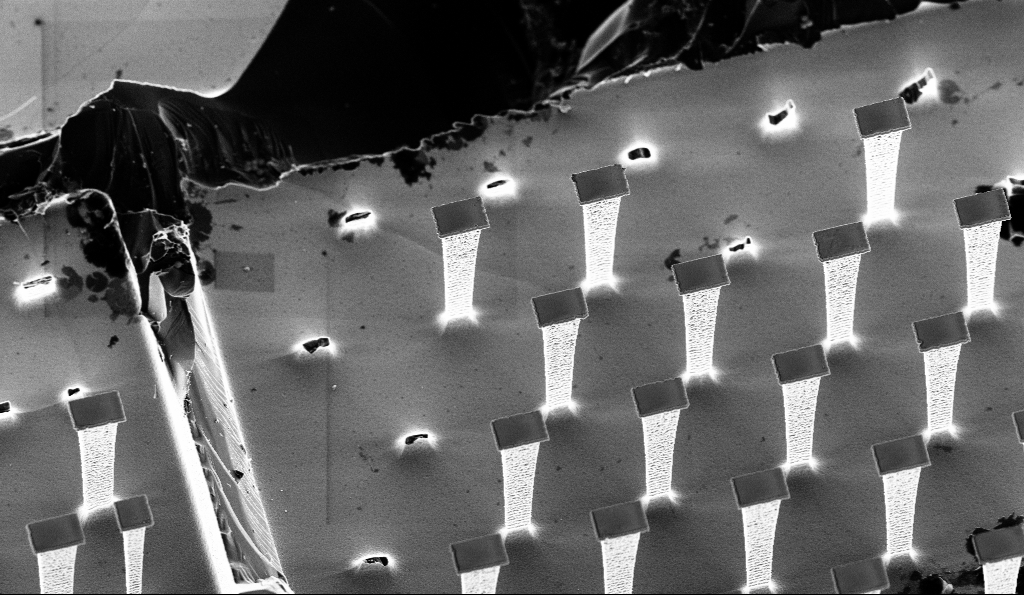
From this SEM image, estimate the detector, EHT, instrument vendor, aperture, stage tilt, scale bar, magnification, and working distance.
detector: InLens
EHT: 3 kV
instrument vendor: Zeiss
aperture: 30 µm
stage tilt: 30°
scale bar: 10000 nm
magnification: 4.4 K X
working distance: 5.6 mm